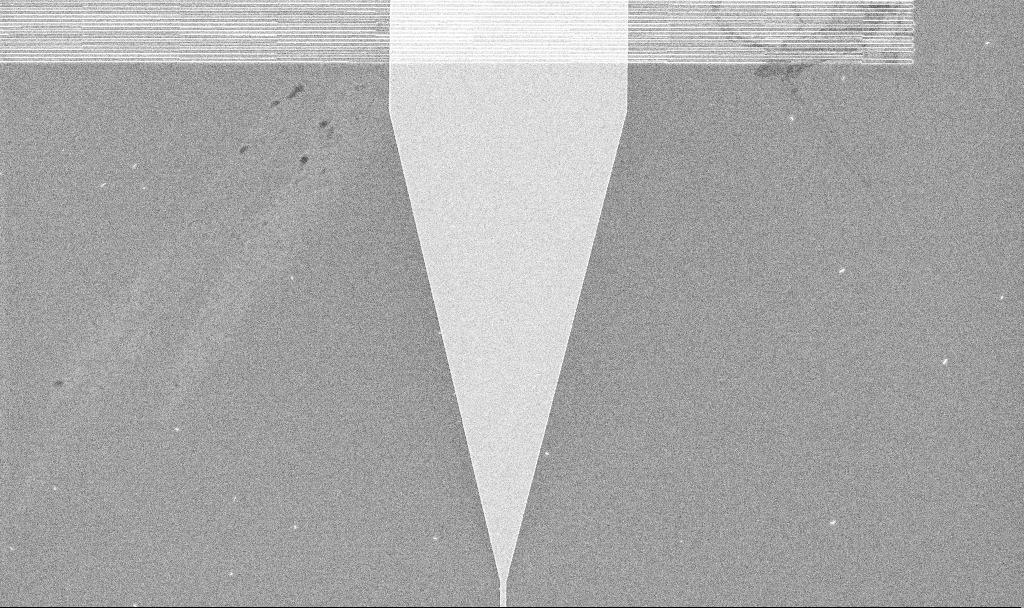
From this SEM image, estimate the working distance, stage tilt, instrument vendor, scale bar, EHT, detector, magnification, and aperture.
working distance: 10.3 mm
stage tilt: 0°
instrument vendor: Zeiss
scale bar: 10000 nm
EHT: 5 kV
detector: InLens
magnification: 4.37 K X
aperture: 30 µm